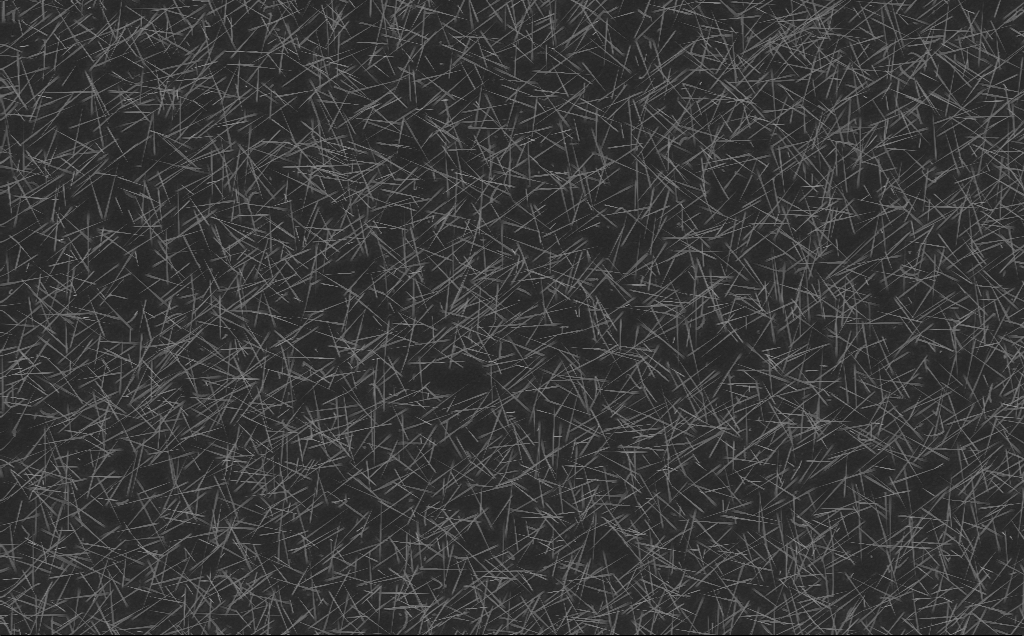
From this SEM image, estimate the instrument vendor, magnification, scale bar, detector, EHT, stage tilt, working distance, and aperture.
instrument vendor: Zeiss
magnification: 5 K X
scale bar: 10000 nm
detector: InLens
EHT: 10 kV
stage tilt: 0°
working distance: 6 mm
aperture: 30 µm